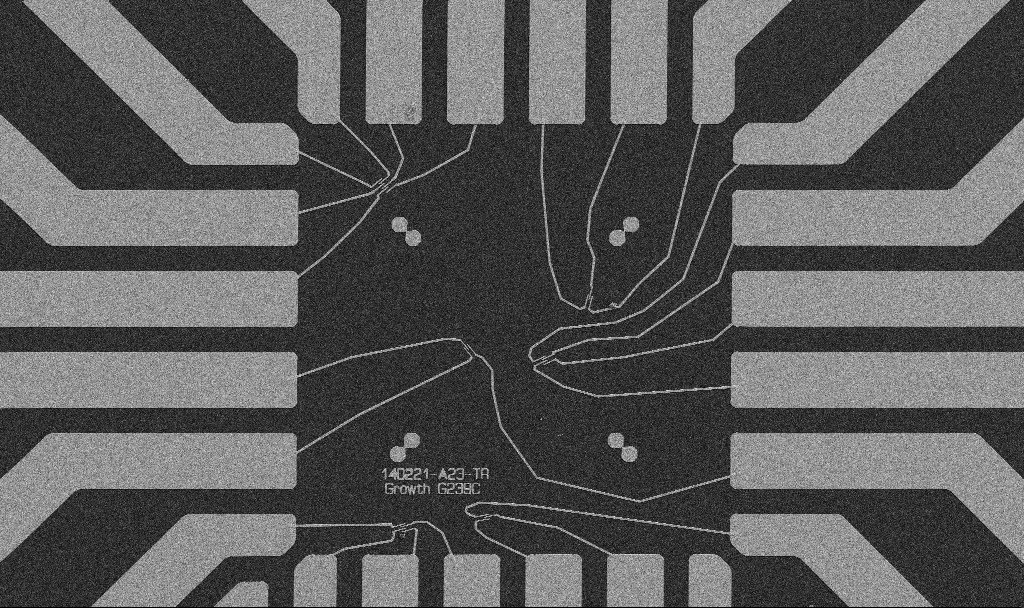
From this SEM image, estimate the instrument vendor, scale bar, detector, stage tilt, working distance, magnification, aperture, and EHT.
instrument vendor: Zeiss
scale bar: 20000 nm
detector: SE2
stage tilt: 0°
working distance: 10.7 mm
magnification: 1 K X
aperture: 30 µm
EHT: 5 kV